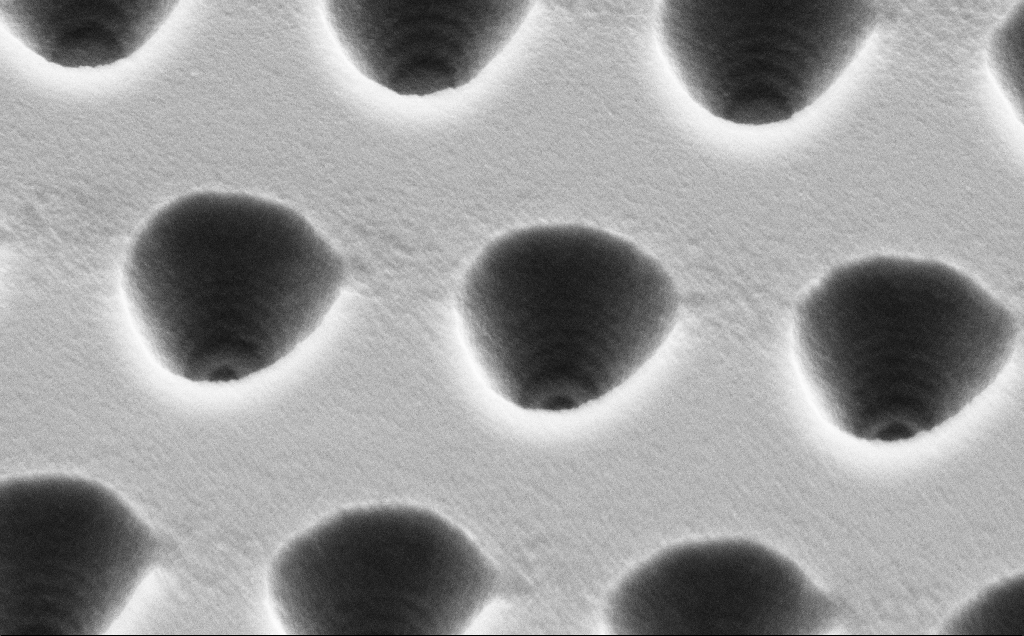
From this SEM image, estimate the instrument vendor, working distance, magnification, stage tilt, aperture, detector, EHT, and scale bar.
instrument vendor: Zeiss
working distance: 10 mm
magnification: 30.67 K X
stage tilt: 45°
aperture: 30 µm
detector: SE2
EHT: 5 kV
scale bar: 2000 nm